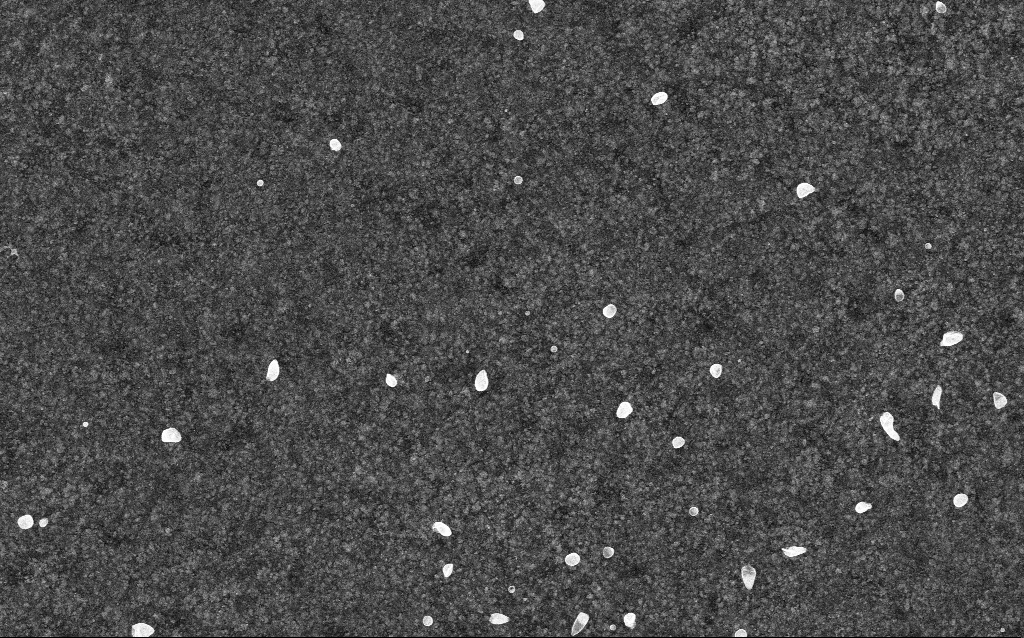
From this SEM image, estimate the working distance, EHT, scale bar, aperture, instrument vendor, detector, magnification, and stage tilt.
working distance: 2.6 mm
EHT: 5 kV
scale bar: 1000 nm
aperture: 30 µm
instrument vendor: Zeiss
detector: InLens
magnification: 50 K X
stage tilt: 0°